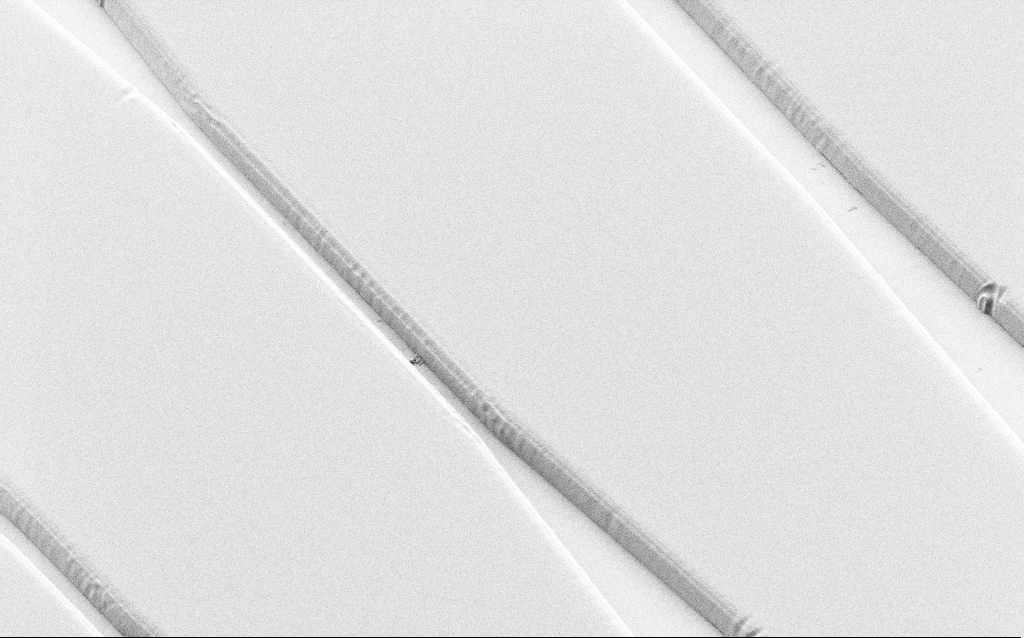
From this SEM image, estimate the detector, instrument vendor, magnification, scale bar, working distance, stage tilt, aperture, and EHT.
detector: SE2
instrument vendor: Zeiss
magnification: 1.57 K X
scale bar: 20000 nm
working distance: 6 mm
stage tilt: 36°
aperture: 30 µm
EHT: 1 kV